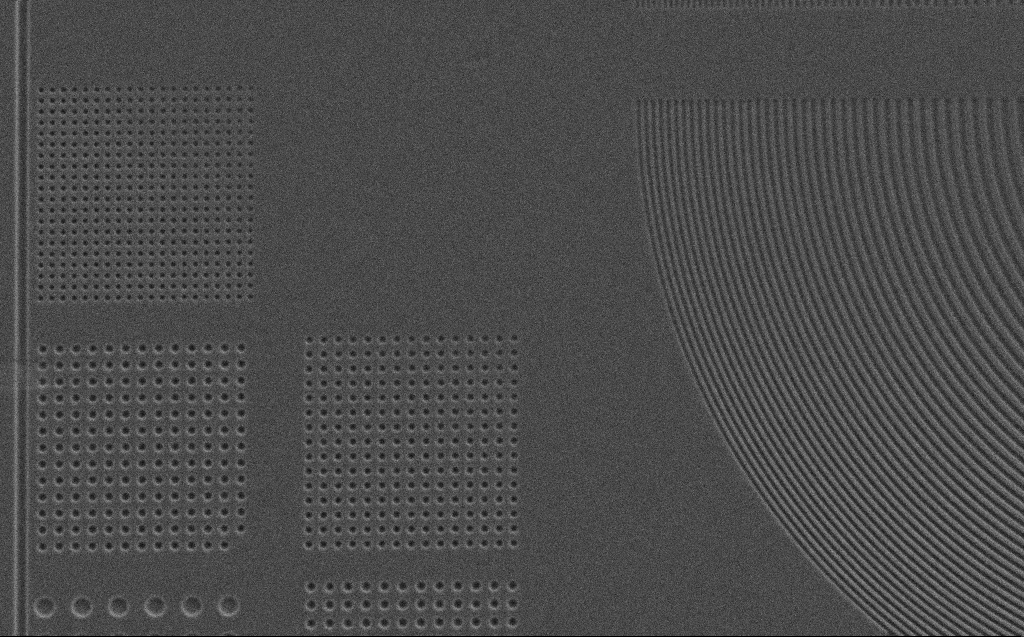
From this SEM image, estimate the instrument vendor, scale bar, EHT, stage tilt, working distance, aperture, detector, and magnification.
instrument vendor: Zeiss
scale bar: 10000 nm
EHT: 5 kV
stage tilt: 0°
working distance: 3 mm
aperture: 30 µm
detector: SE2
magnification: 3.41 K X